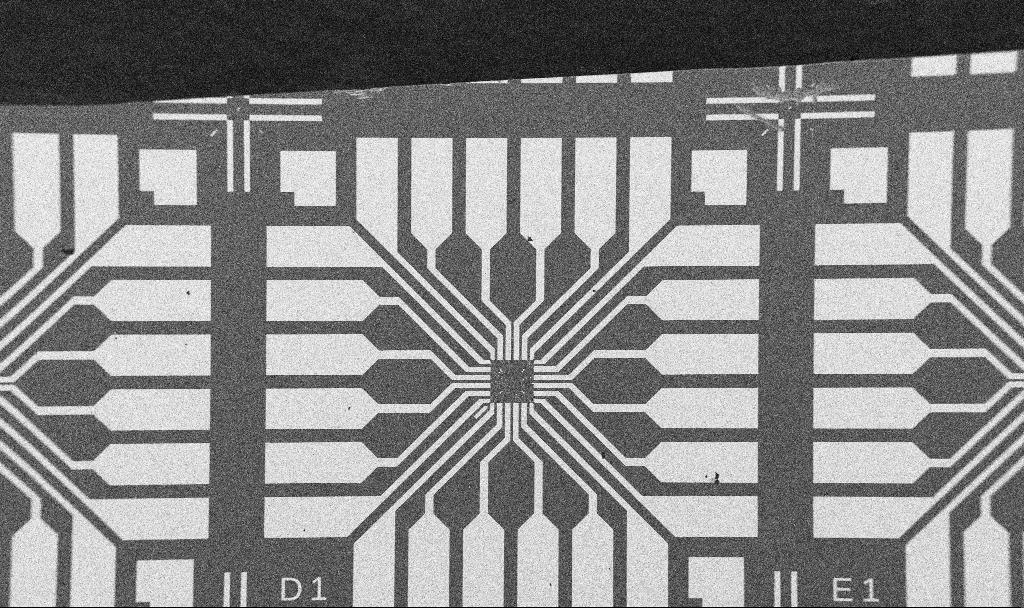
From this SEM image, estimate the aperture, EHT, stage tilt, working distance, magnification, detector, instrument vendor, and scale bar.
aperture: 30 µm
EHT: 5 kV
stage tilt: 0°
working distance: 10.7 mm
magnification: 0.1 K X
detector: SE2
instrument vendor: Zeiss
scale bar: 200000 nm